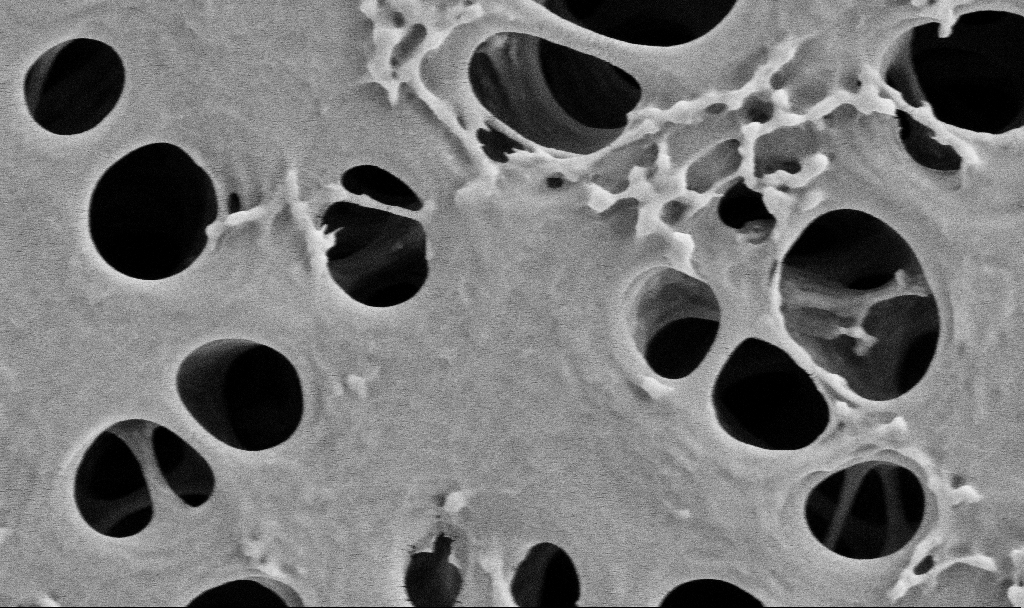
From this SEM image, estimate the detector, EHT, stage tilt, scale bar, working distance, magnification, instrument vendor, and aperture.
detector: SE2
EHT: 2 kV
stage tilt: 0°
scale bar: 1000 nm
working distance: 3.7 mm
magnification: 50 K X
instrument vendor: Zeiss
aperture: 30 µm